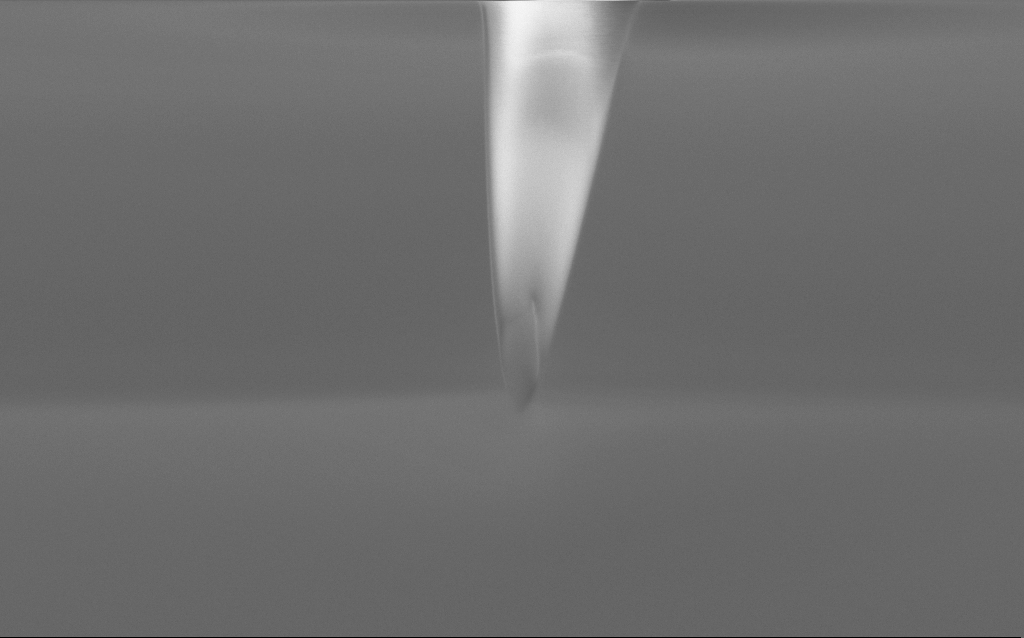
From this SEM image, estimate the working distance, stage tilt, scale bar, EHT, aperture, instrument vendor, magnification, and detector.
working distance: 5 mm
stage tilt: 45°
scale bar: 1000 nm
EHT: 2 kV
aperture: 30 µm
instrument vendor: Zeiss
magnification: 57.83 K X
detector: InLens